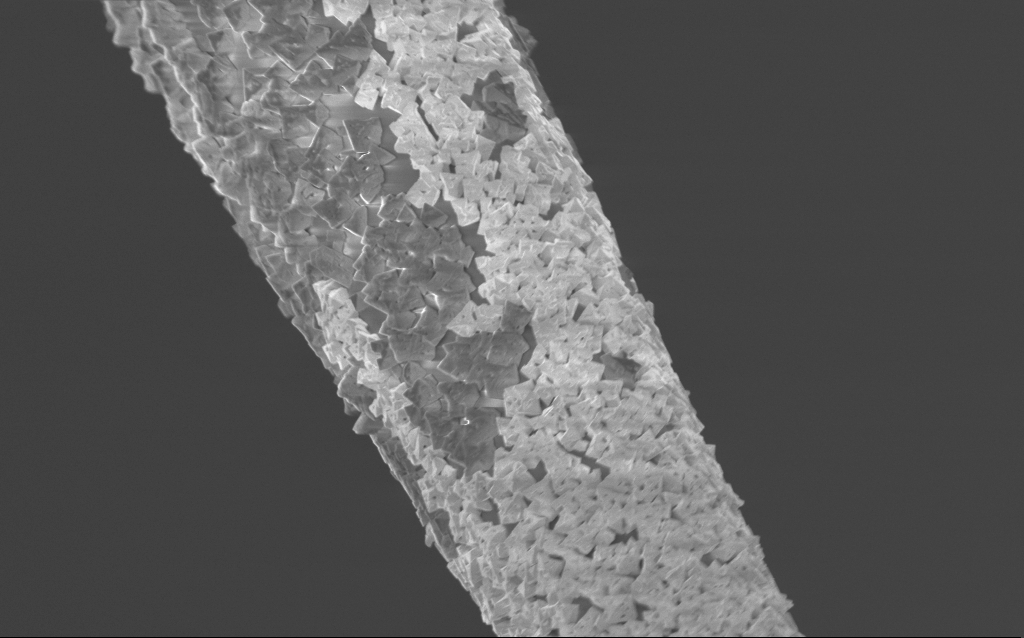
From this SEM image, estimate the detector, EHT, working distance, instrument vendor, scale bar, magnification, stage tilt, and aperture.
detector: InLens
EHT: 1.5 kV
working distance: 7.6 mm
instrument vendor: Zeiss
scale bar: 2000 nm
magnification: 10 K X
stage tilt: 45°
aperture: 30 µm